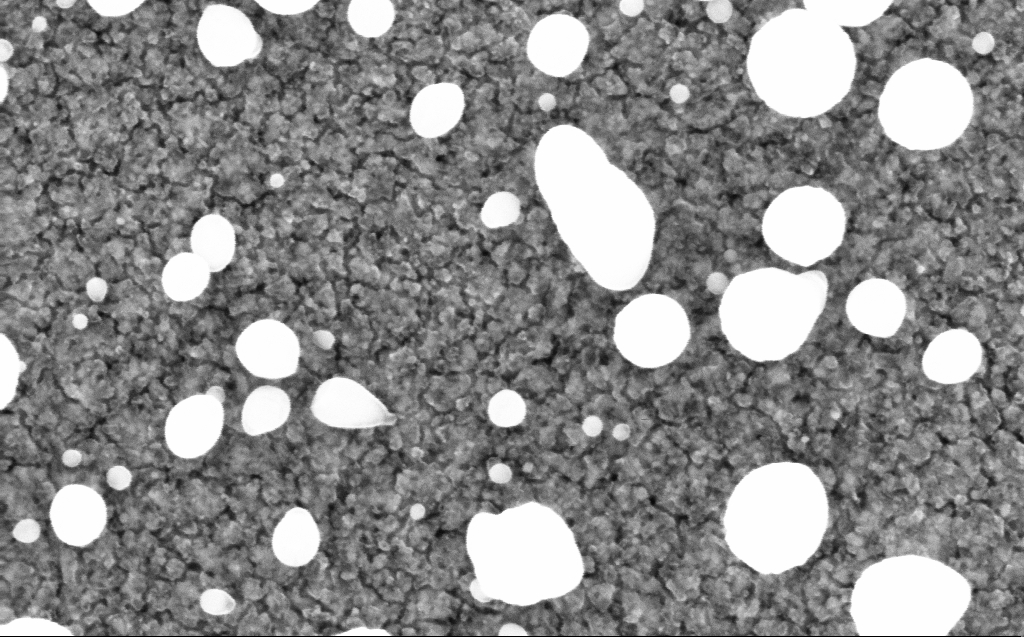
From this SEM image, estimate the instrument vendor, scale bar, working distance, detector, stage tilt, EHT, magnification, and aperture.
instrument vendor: Zeiss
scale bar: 100 nm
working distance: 3 mm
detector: InLens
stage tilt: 0°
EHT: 10 kV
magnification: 200 K X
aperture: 30 µm